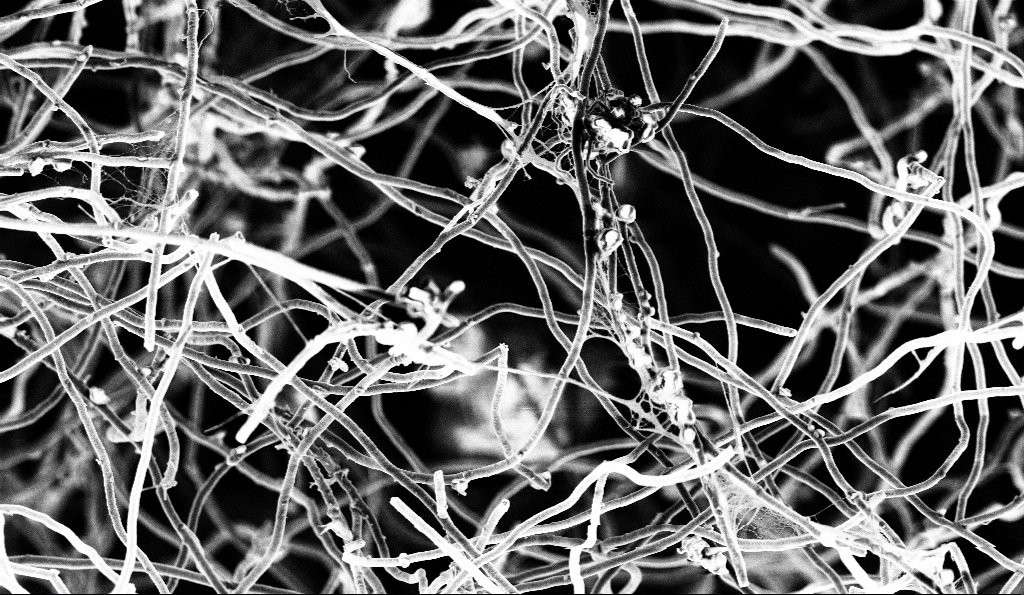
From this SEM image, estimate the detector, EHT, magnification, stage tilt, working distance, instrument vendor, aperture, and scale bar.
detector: InLens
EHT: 3 kV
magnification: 5 K X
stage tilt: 0°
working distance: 5 mm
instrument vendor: Zeiss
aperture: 30 µm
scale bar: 10000 nm